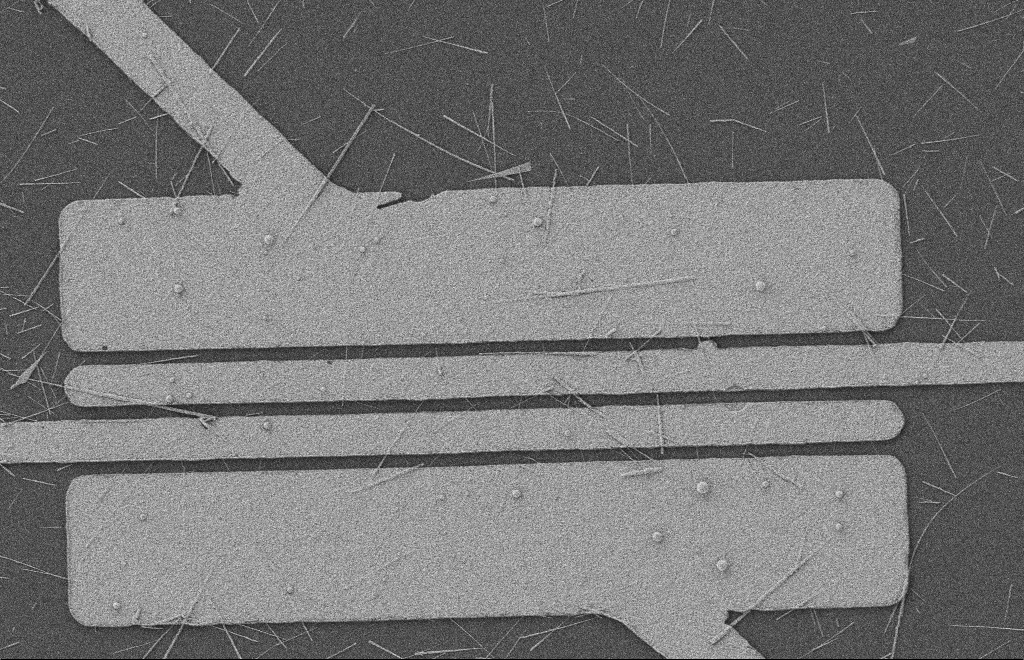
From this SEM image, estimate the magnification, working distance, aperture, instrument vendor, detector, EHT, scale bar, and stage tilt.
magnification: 5.03 K X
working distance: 8 mm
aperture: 20 µm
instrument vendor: Zeiss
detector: SE2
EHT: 2 kV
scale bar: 2000 nm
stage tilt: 0°